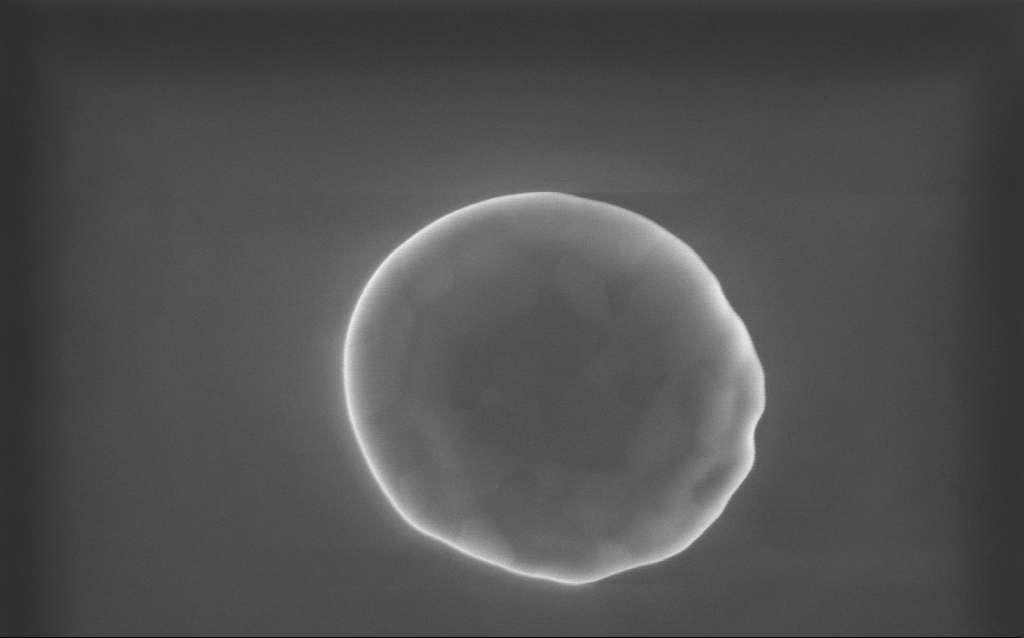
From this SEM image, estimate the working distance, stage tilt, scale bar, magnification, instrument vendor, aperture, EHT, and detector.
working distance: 2 mm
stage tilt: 0°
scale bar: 1000 nm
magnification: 63 K X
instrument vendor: Zeiss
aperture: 30 µm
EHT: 10 kV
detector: InLens